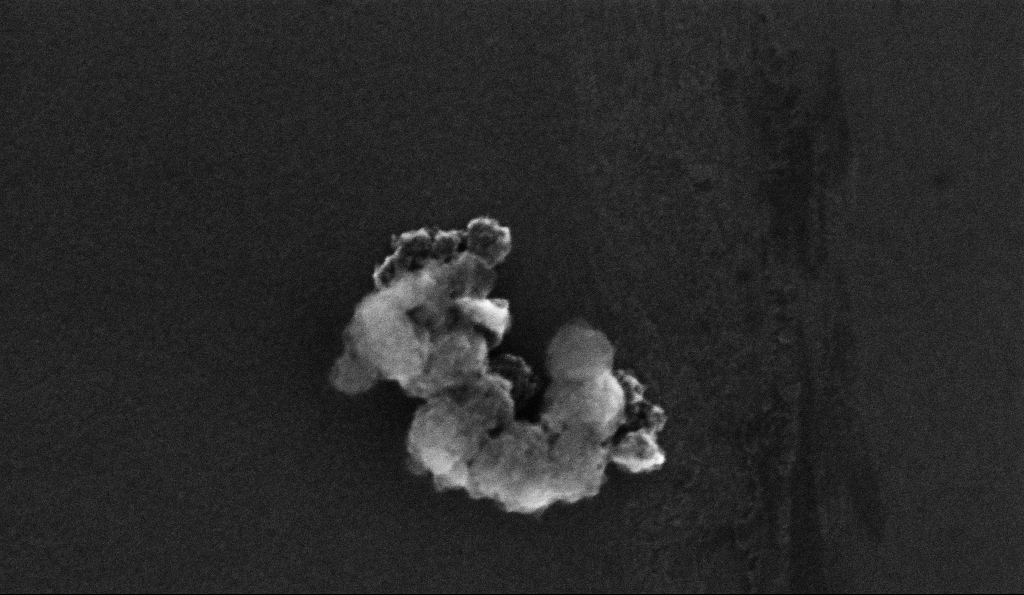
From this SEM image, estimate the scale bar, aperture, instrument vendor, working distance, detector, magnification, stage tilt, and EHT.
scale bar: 200 nm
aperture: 30 µm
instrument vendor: Zeiss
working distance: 5.3 mm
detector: InLens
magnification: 301.98 K X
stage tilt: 0°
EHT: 10 kV